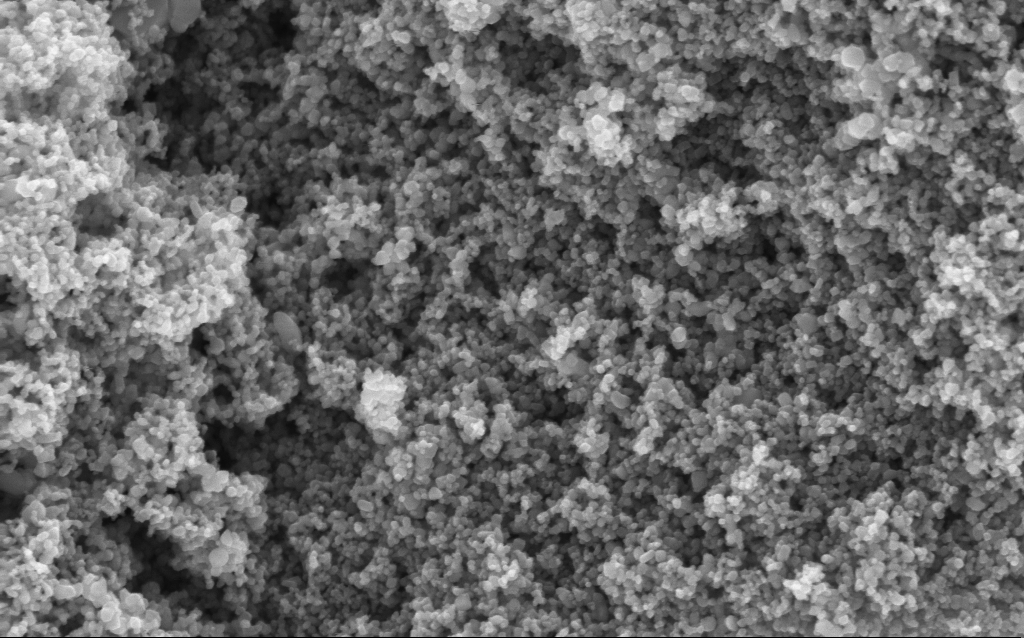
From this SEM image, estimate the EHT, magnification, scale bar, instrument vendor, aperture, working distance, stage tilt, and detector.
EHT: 5 kV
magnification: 114.65 K X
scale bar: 200 nm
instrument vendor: Zeiss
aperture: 30 µm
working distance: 4.5 mm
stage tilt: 0°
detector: InLens